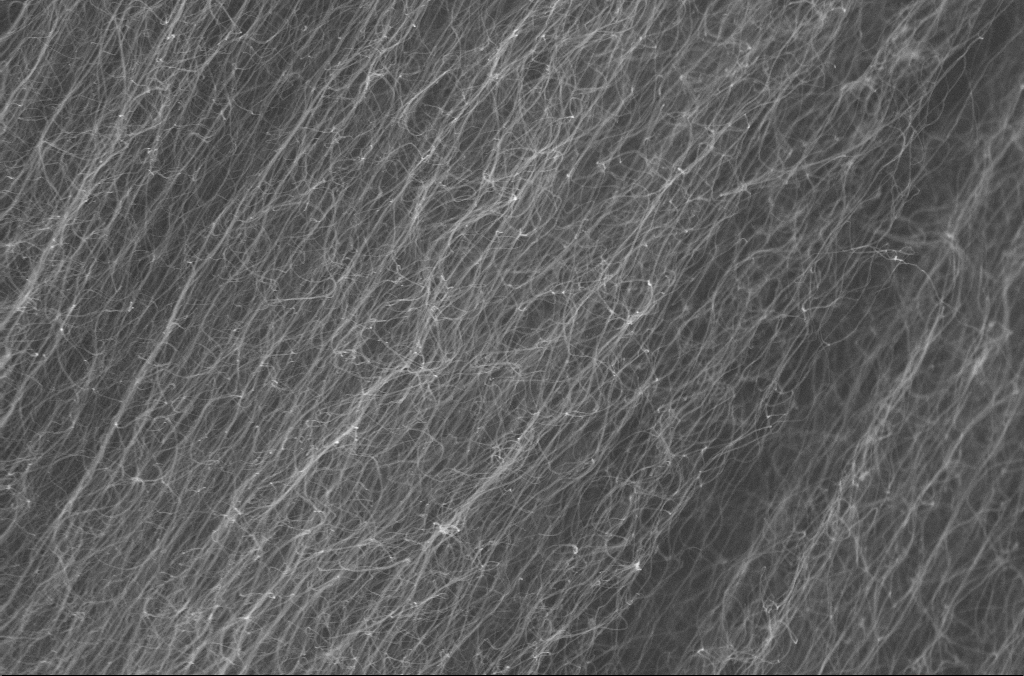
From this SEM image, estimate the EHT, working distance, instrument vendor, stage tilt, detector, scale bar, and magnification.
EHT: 10 kV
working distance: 4 mm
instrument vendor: Zeiss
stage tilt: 0°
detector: InLens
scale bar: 1000 nm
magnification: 45 K X